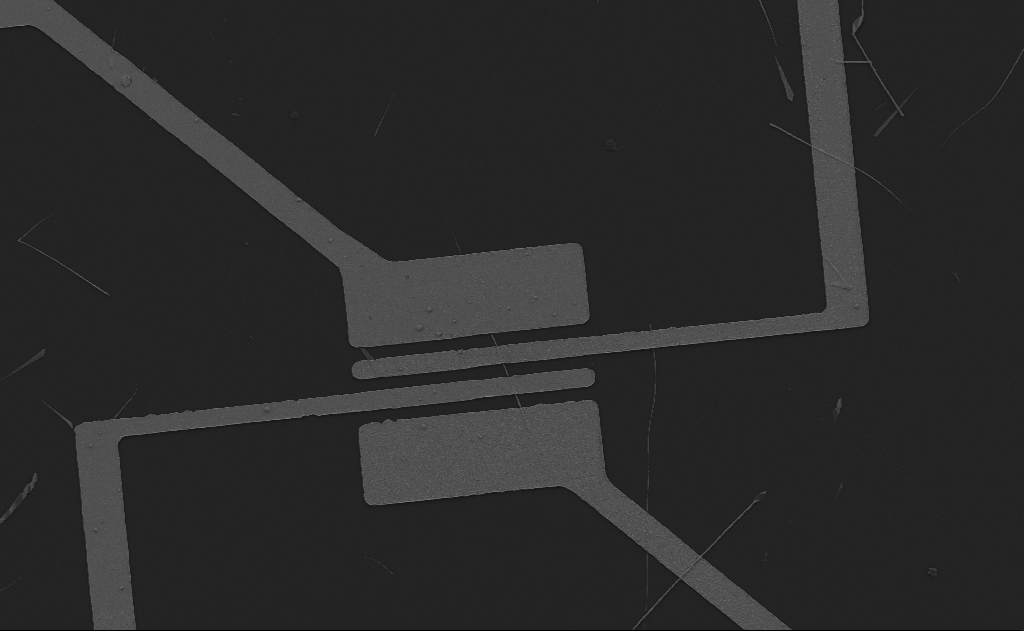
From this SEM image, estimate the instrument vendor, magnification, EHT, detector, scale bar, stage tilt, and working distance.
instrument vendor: Zeiss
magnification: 2.93 K X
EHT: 5 kV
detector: SE2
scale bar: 10000 nm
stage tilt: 0°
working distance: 13 mm